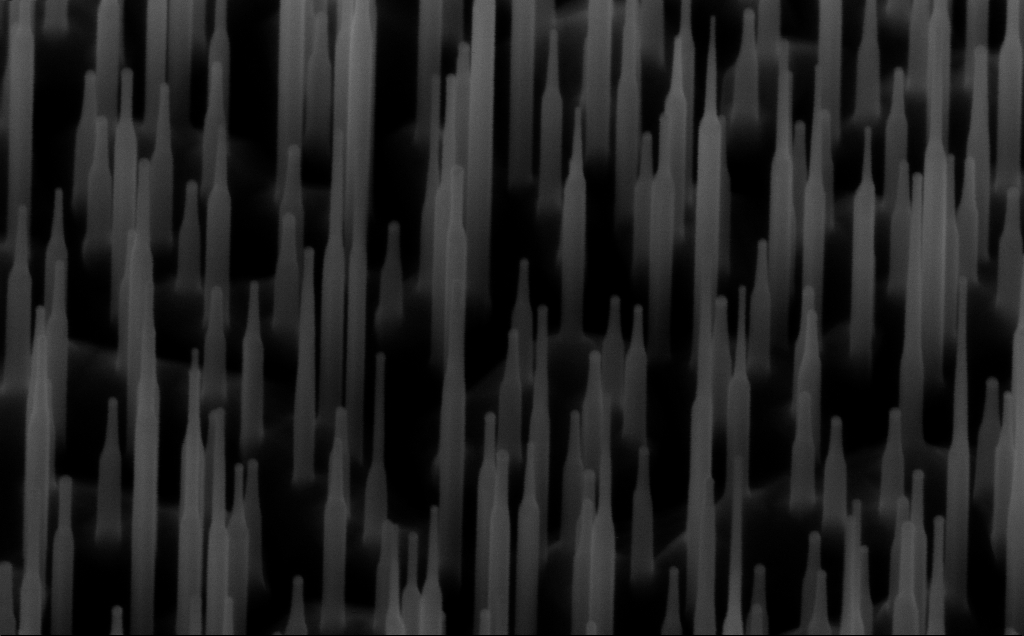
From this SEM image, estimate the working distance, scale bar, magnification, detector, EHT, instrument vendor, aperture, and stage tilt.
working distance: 6 mm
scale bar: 100 nm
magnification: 150 K X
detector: InLens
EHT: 10 kV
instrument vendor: Zeiss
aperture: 30 µm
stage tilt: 45°